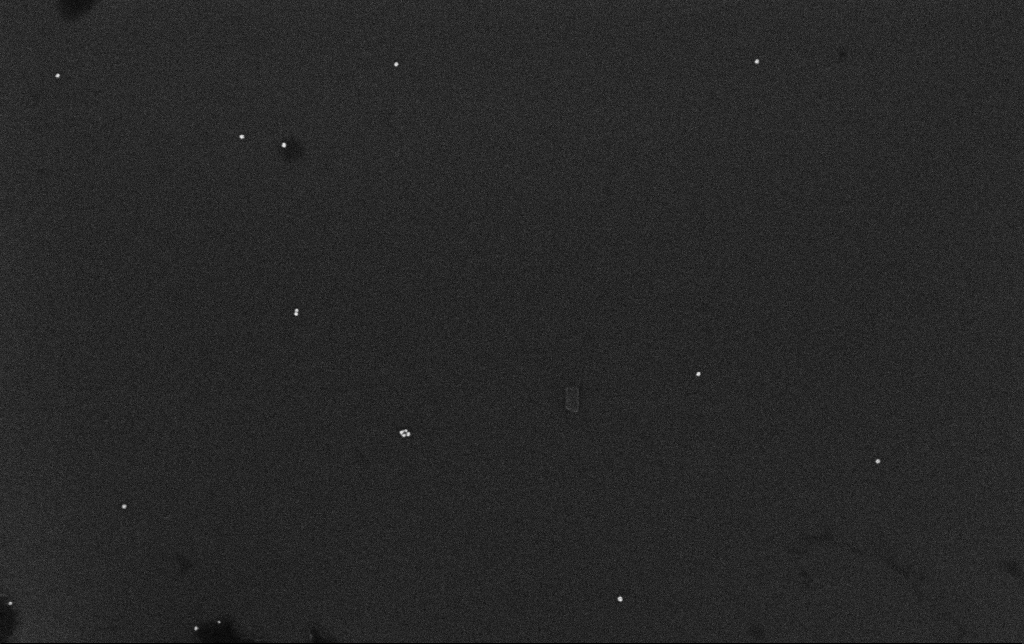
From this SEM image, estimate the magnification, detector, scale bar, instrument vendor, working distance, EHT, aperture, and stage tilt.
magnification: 100 K X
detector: InLens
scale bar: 200 nm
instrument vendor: Zeiss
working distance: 3.2 mm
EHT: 10 kV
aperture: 30 µm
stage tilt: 0°